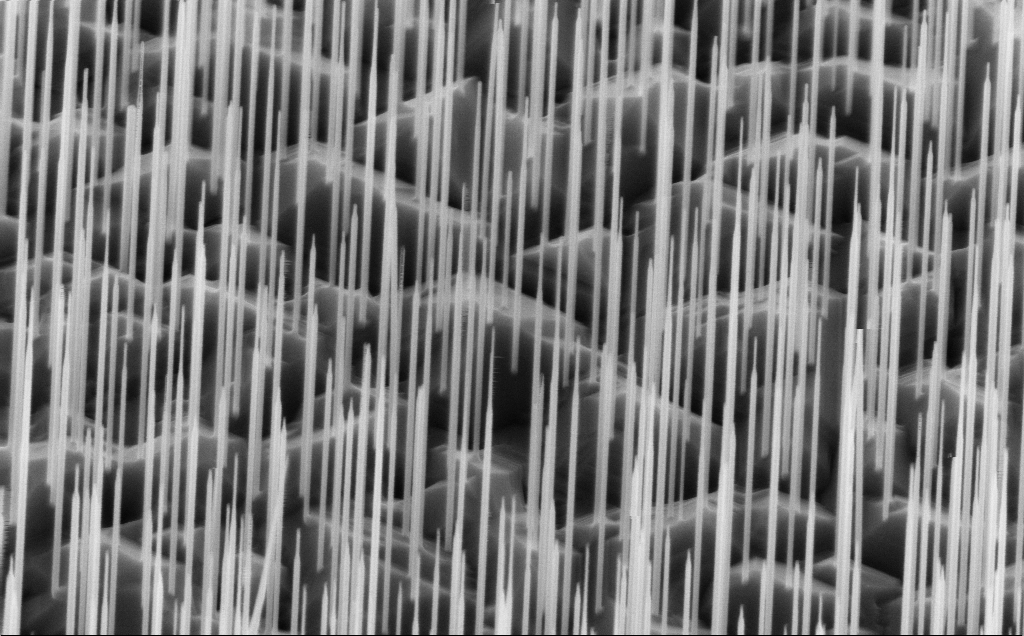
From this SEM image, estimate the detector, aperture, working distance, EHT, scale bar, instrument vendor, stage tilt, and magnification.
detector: InLens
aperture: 30 µm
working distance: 5 mm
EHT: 10 kV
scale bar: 1000 nm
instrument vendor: Zeiss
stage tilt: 45°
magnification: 40 K X